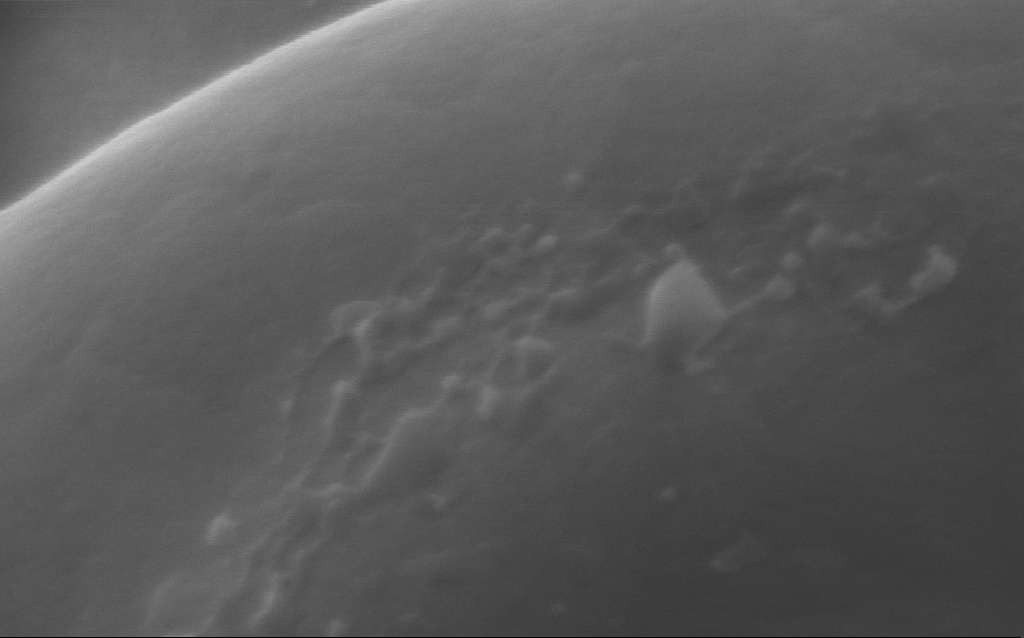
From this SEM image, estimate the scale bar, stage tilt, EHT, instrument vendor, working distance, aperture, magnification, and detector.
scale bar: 200 nm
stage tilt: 0°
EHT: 5 kV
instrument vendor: Zeiss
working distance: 3 mm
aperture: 30 µm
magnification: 254 K X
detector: InLens